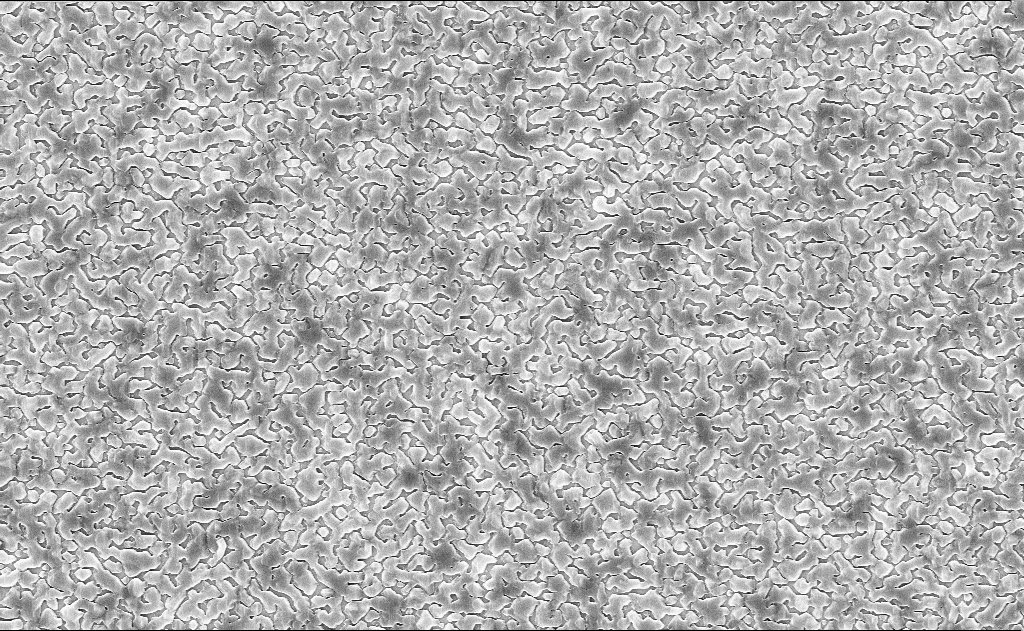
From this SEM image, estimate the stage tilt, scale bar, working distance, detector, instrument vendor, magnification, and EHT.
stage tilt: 0°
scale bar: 2000 nm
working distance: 11 mm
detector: InLens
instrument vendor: Zeiss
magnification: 20 K X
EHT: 10 kV